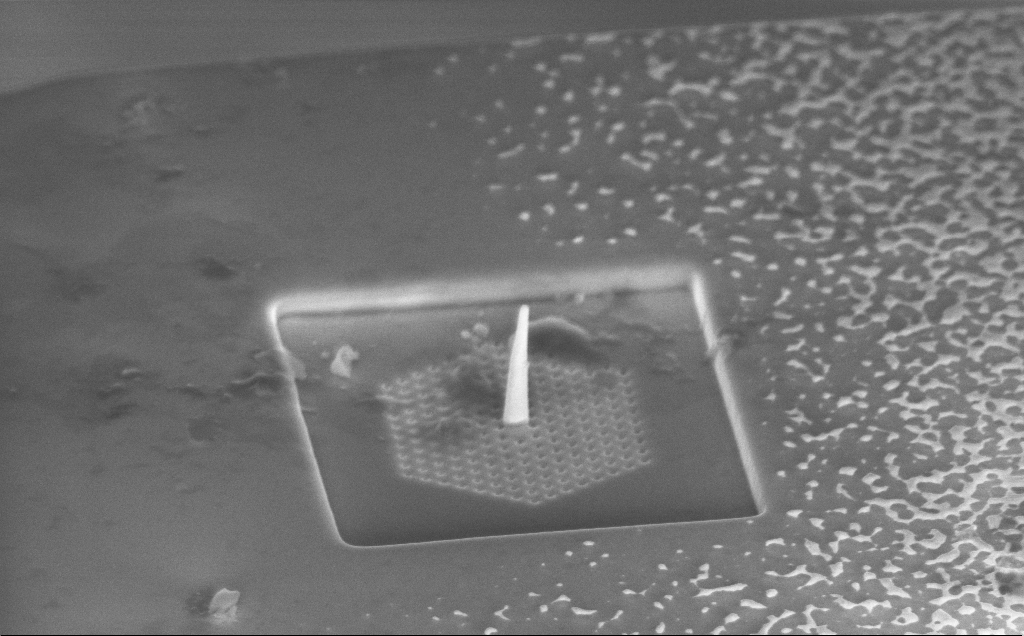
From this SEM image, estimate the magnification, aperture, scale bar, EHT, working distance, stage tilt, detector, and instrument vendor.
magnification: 25.28 K X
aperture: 30 µm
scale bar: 1000 nm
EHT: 5 kV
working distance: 6 mm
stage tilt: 53.9°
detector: InLens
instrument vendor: Zeiss